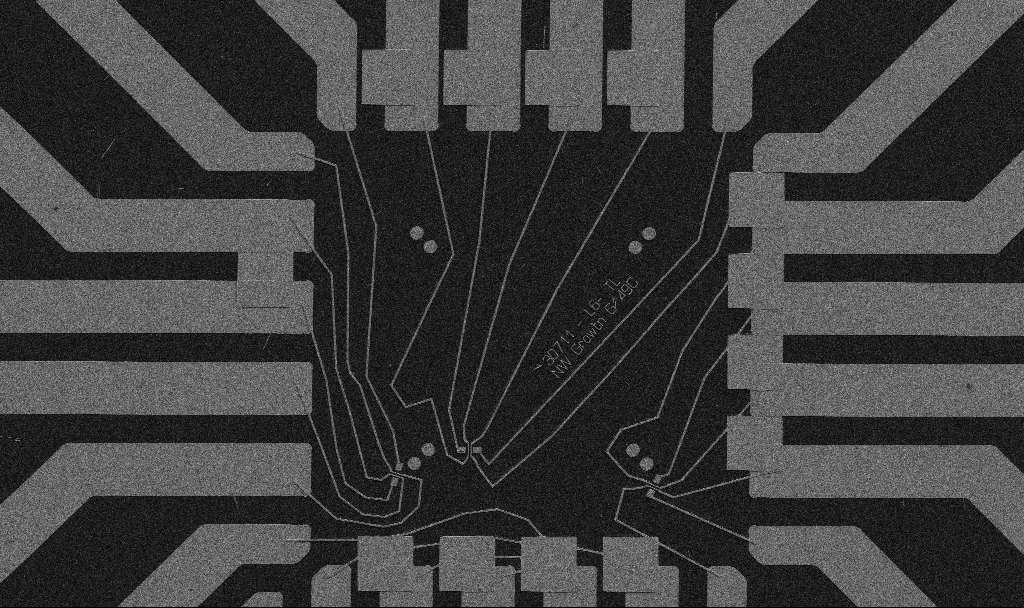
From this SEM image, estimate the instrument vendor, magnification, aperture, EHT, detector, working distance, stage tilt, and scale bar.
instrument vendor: Zeiss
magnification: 1 K X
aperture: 30 µm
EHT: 5 kV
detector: SE2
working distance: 10.7 mm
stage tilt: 0°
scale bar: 20000 nm